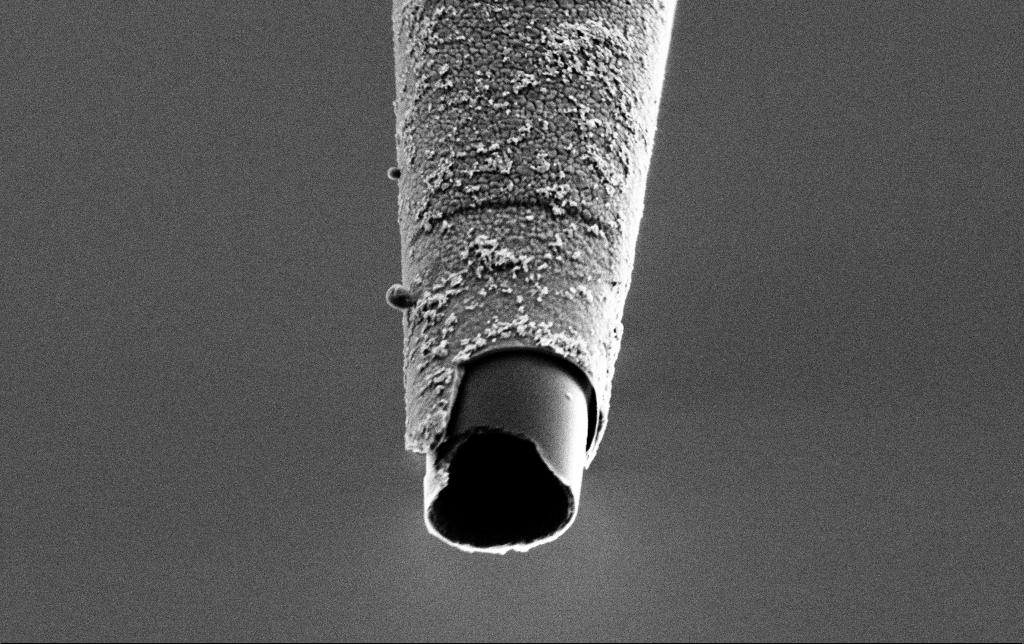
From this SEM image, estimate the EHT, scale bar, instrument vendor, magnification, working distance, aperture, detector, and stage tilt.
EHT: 2 kV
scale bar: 1000 nm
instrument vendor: Zeiss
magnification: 25 K X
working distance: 7.6 mm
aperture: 30 µm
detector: SE2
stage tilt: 45°